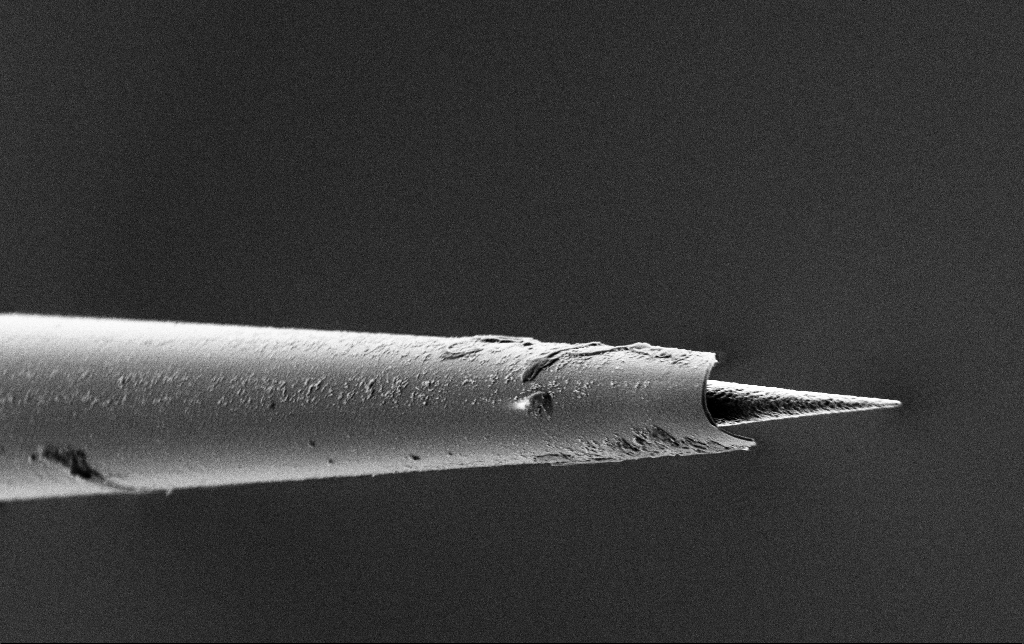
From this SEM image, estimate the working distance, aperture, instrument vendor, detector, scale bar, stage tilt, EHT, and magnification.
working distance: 7.4 mm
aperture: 30 µm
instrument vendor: Zeiss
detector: SE2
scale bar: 2000 nm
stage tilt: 45°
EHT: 2 kV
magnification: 10 K X